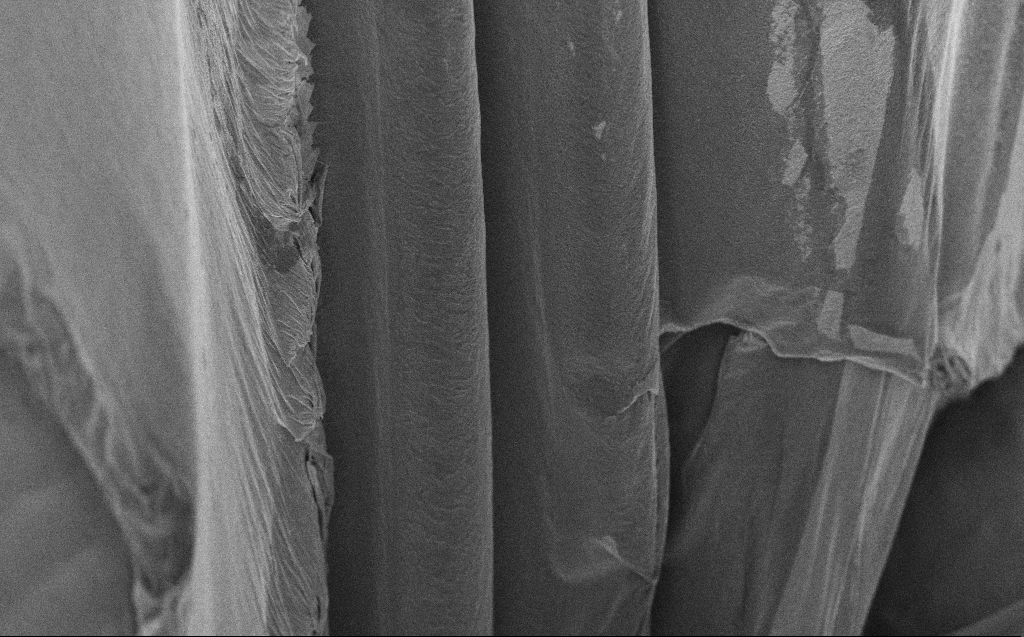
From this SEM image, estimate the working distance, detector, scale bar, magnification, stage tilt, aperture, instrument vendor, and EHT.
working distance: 9 mm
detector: InLens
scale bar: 10000 nm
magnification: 5.97 K X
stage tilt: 45°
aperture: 30 µm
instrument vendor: Zeiss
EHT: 5 kV